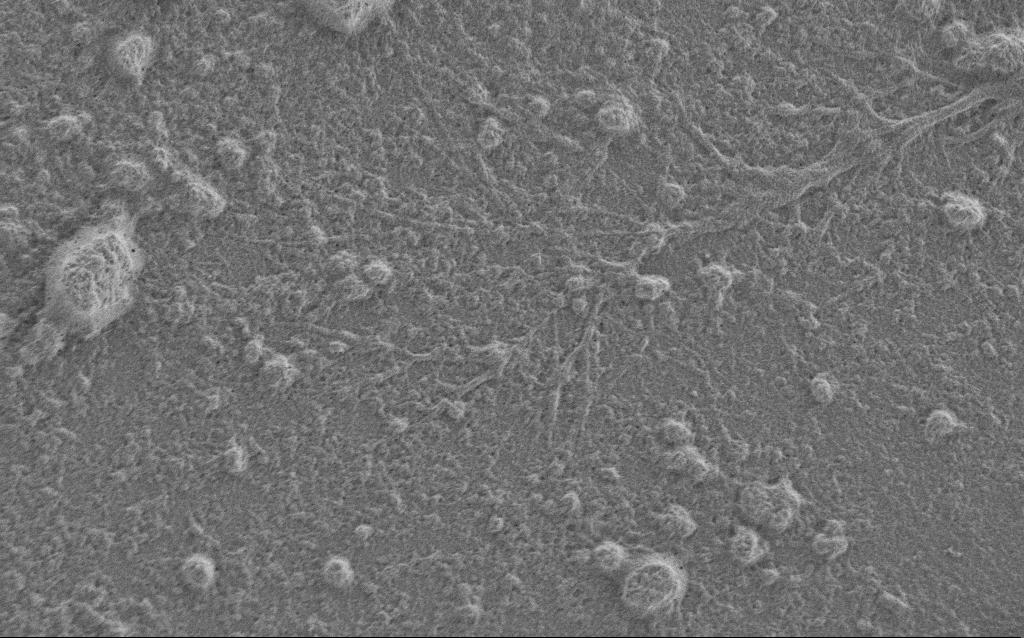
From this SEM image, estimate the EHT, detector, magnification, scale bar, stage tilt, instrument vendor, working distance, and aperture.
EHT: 1 kV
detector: SE2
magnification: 7.5 K X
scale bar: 2000 nm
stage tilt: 0°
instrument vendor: Zeiss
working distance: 6 mm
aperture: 30 µm